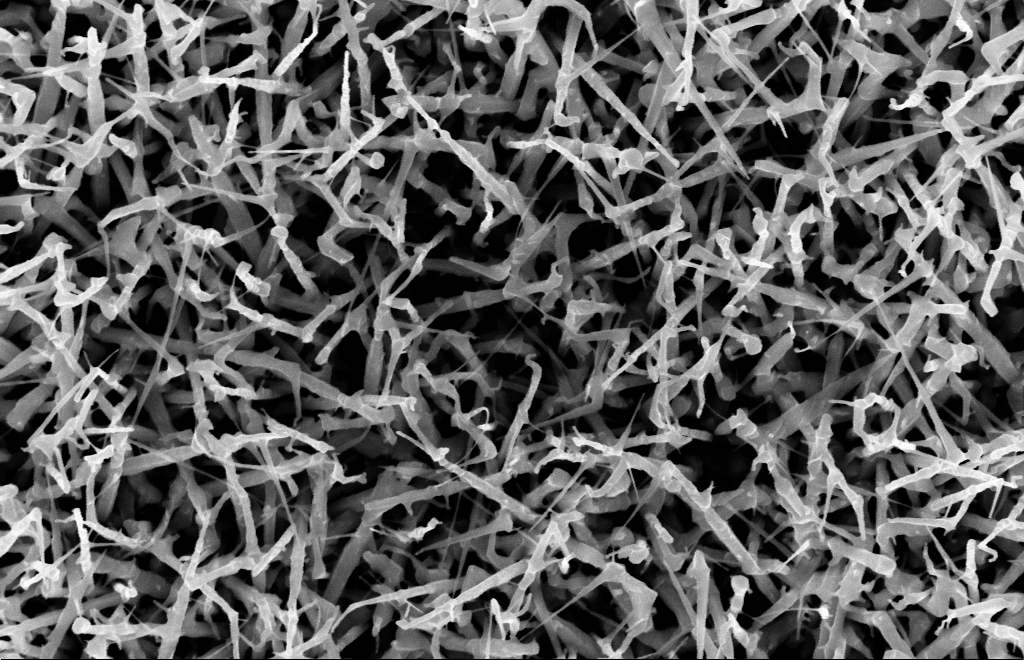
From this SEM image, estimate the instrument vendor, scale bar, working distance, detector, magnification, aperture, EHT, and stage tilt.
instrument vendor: Zeiss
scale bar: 1000 nm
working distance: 15 mm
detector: InLens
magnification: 40 K X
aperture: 30 µm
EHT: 10 kV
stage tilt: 0°